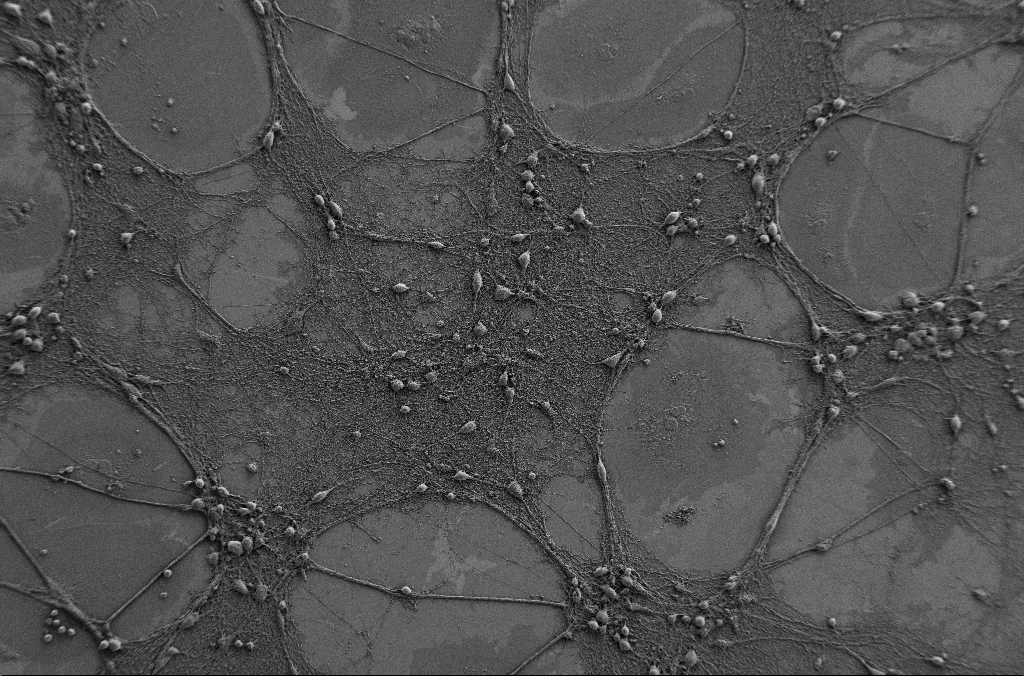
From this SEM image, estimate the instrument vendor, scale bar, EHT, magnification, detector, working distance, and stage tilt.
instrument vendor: Zeiss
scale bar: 100000 nm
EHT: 1 kV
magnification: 0.5 K X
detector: SE2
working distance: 4.1 mm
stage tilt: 0°